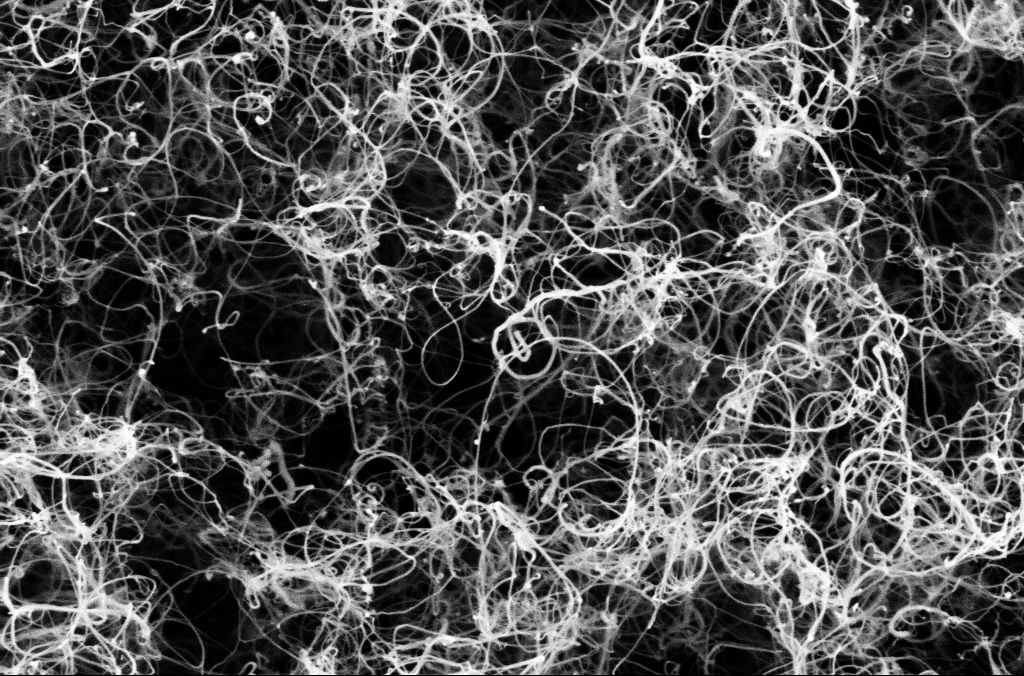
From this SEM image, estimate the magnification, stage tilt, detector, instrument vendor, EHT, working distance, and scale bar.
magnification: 50 K X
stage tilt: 0°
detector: SE2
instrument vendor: Zeiss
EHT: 1.8 kV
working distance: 5.3 mm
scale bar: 1000 nm